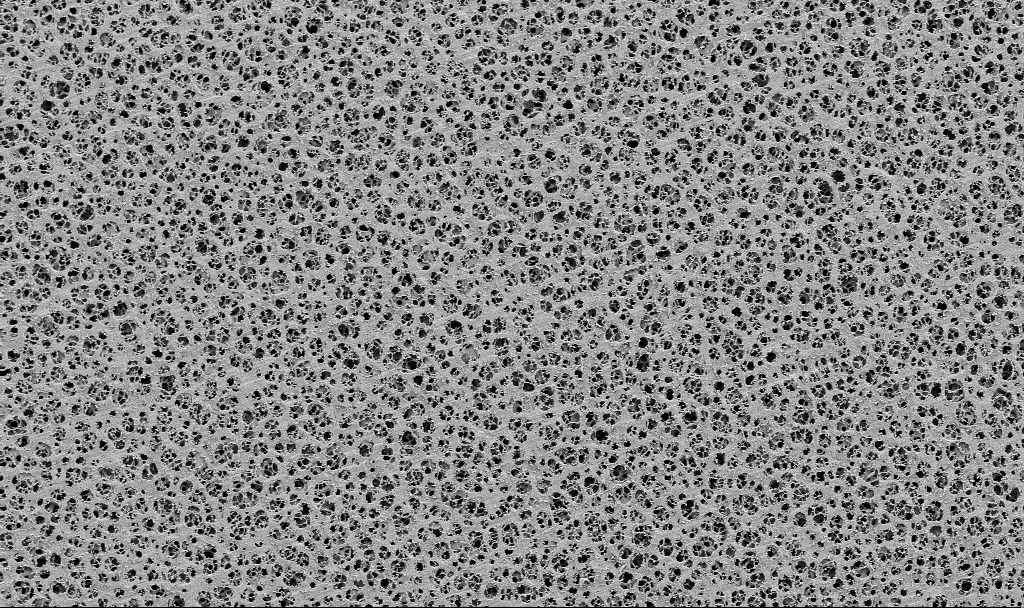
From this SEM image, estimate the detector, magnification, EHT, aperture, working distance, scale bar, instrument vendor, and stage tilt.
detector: SE2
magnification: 2 K X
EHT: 2 kV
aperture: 30 µm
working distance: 3.5 mm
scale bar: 10000 nm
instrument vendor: Zeiss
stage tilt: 0°